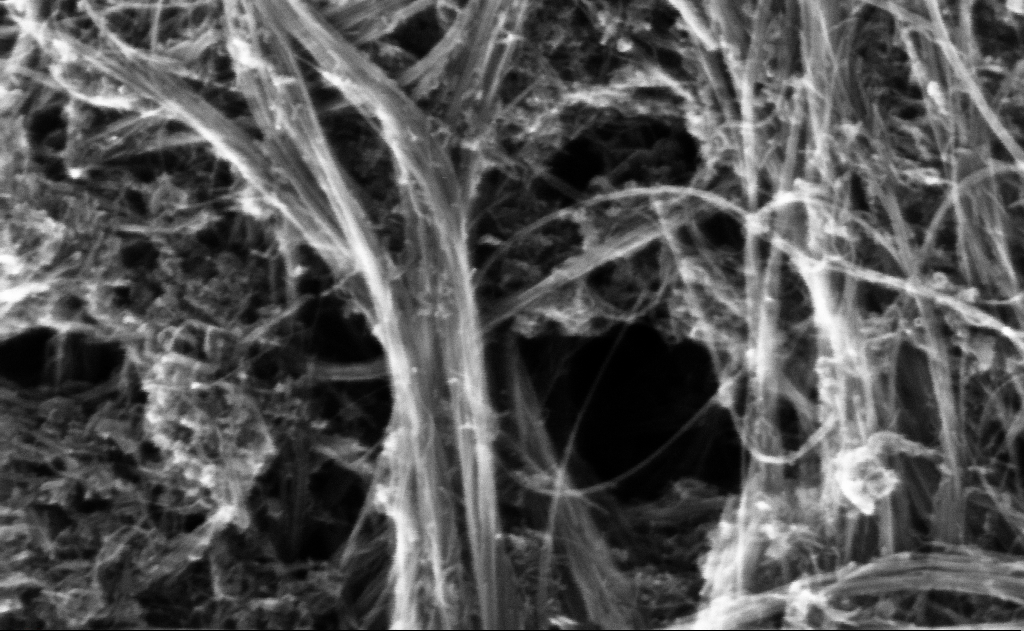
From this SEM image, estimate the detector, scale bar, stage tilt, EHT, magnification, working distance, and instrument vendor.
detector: InLens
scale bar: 100 nm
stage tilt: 0°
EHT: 10 kV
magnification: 372.83 K X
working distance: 5 mm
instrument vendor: Zeiss